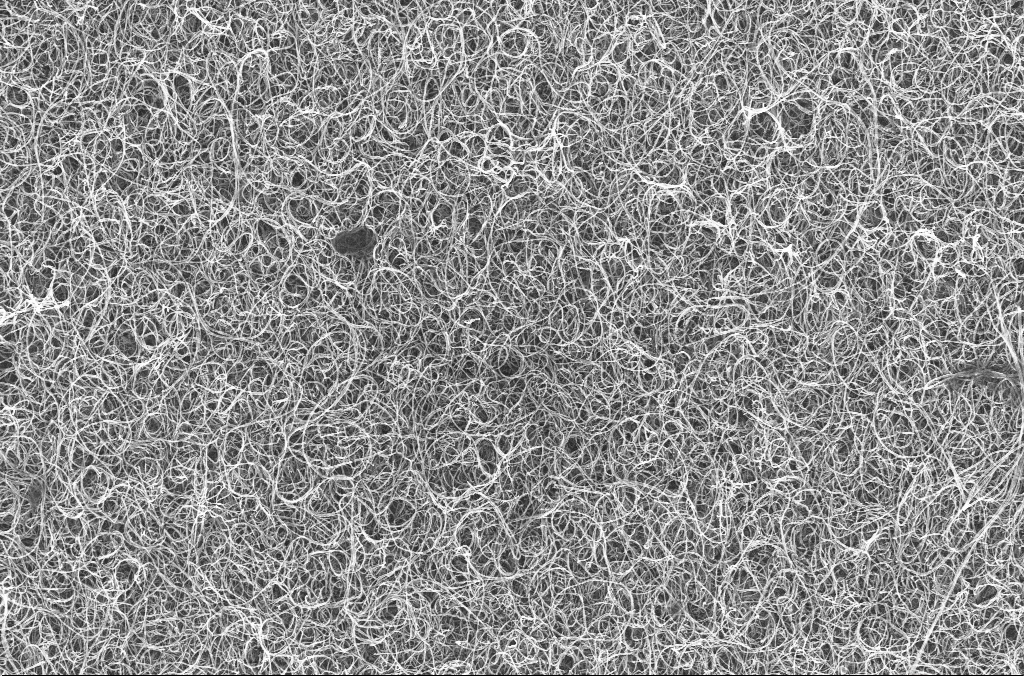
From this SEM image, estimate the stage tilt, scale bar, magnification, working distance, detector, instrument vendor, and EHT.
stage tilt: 0°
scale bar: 1000 nm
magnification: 15 K X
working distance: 4.5 mm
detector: InLens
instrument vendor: Zeiss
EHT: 20 kV